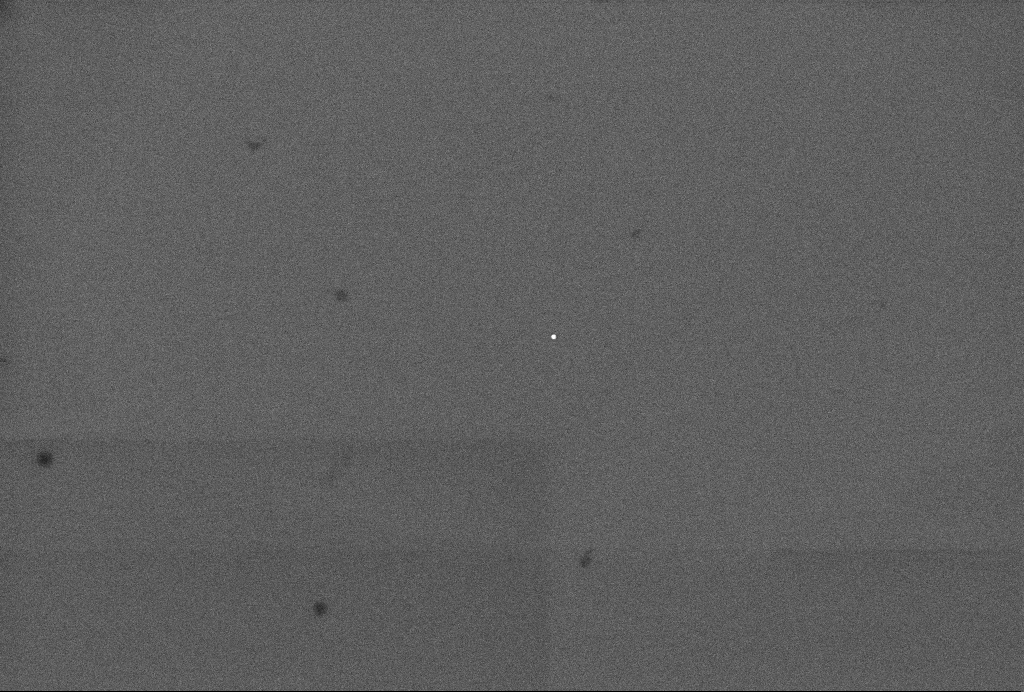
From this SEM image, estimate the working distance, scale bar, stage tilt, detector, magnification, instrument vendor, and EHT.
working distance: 3.3 mm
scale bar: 200 nm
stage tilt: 0°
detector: InLens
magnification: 68.71 K X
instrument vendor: Zeiss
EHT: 3 kV